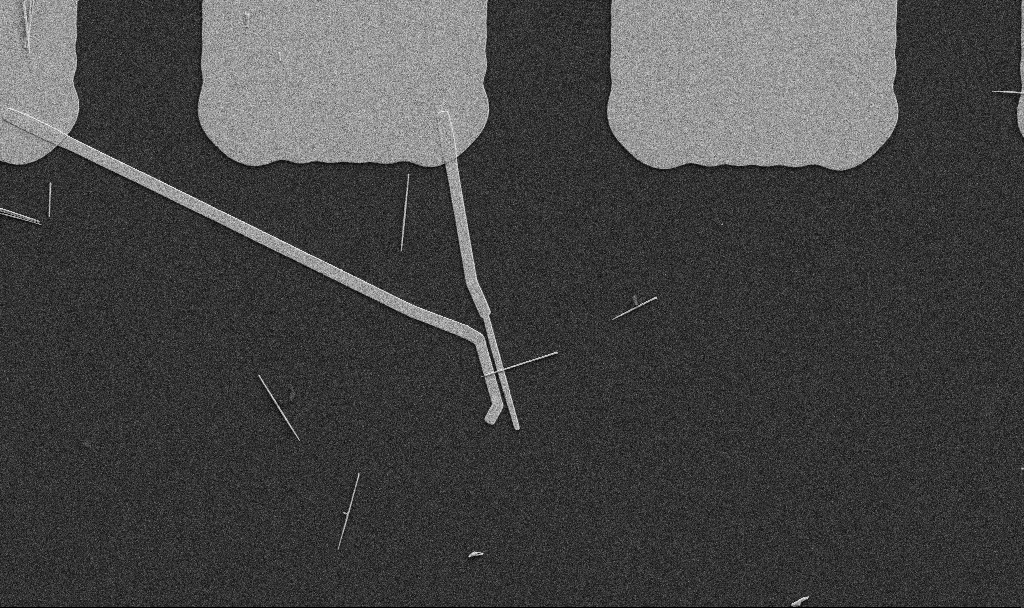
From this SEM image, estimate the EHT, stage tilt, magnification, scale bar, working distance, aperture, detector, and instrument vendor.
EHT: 5 kV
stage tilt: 0°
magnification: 5 K X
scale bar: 10000 nm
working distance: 10.7 mm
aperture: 30 µm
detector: SE2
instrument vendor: Zeiss